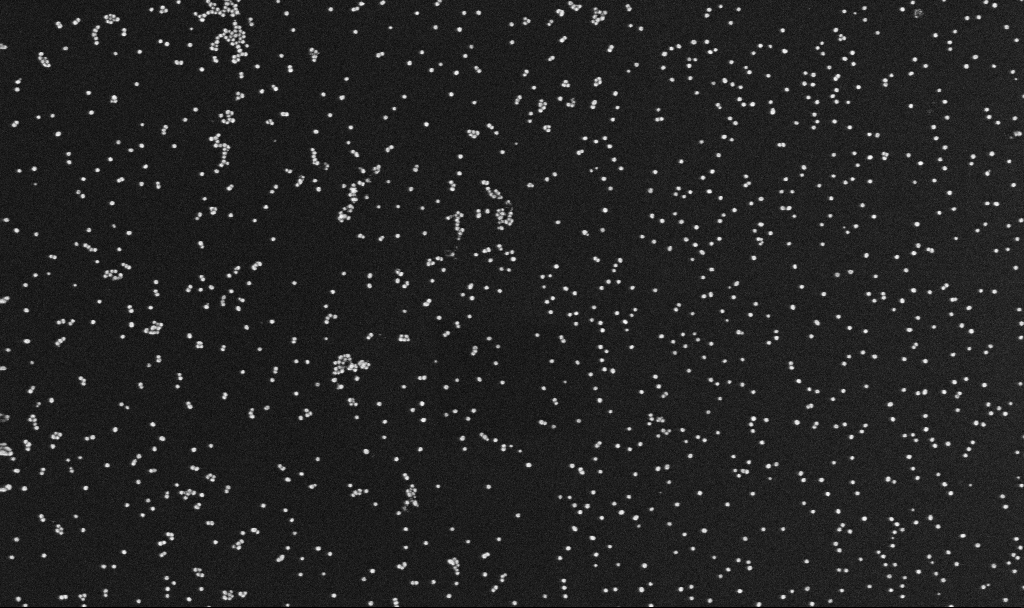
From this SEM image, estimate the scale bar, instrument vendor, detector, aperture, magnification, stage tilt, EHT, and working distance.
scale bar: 200 nm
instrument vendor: Zeiss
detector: InLens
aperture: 30 µm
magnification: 100 K X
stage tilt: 0°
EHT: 10 kV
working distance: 3.4 mm